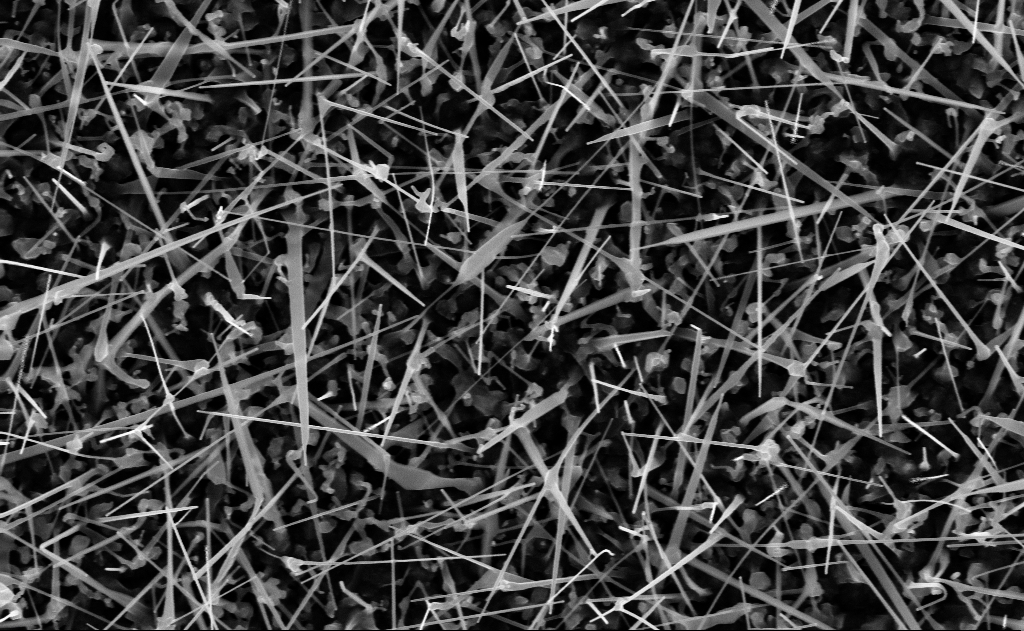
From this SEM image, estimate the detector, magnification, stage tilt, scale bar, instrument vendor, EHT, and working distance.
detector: InLens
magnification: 40 K X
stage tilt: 0°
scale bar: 1000 nm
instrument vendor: Zeiss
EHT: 10 kV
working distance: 12 mm